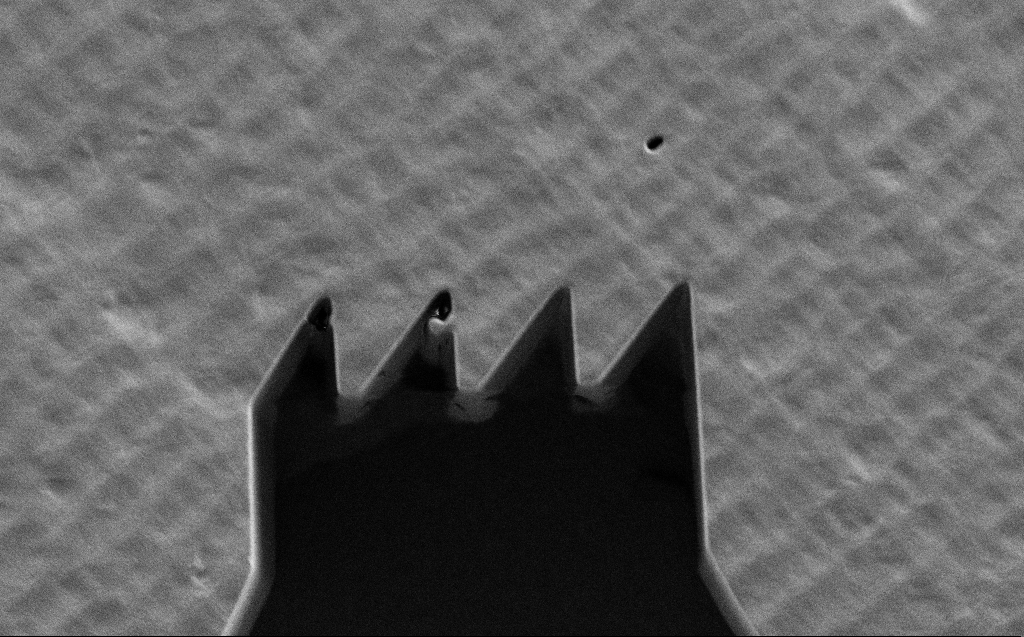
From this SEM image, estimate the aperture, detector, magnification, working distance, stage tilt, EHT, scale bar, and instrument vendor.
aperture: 30 µm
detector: SE2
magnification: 3.81 K X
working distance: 6 mm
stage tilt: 0°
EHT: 1 kV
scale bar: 10000 nm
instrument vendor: Zeiss